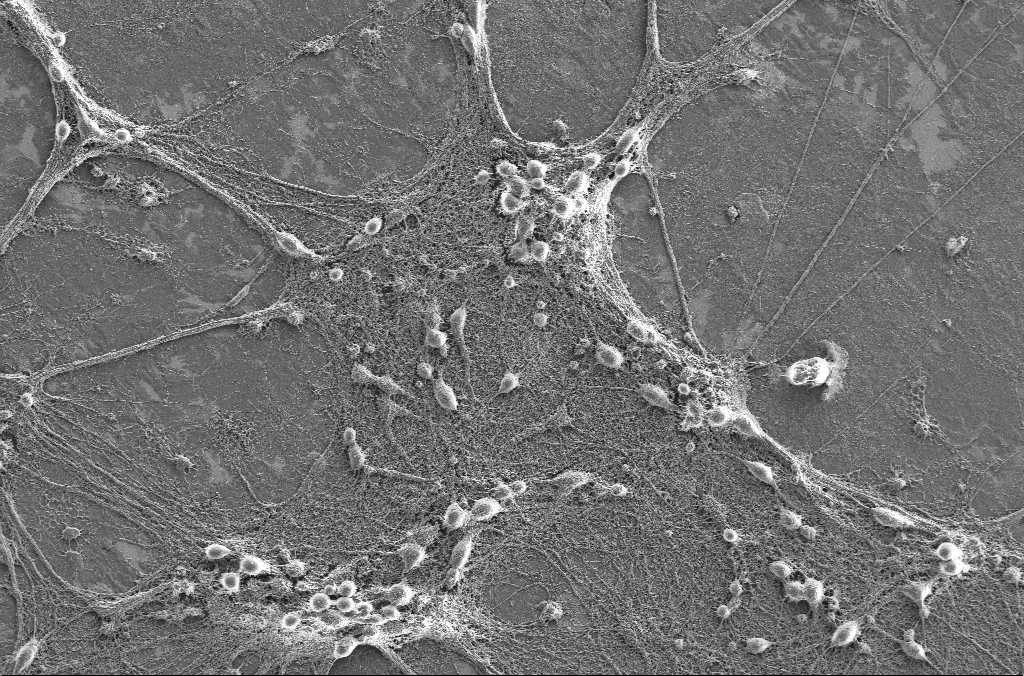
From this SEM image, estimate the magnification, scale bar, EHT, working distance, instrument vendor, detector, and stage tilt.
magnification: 1 K X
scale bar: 20000 nm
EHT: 2 kV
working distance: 4.1 mm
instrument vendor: Zeiss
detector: SE2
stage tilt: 0°